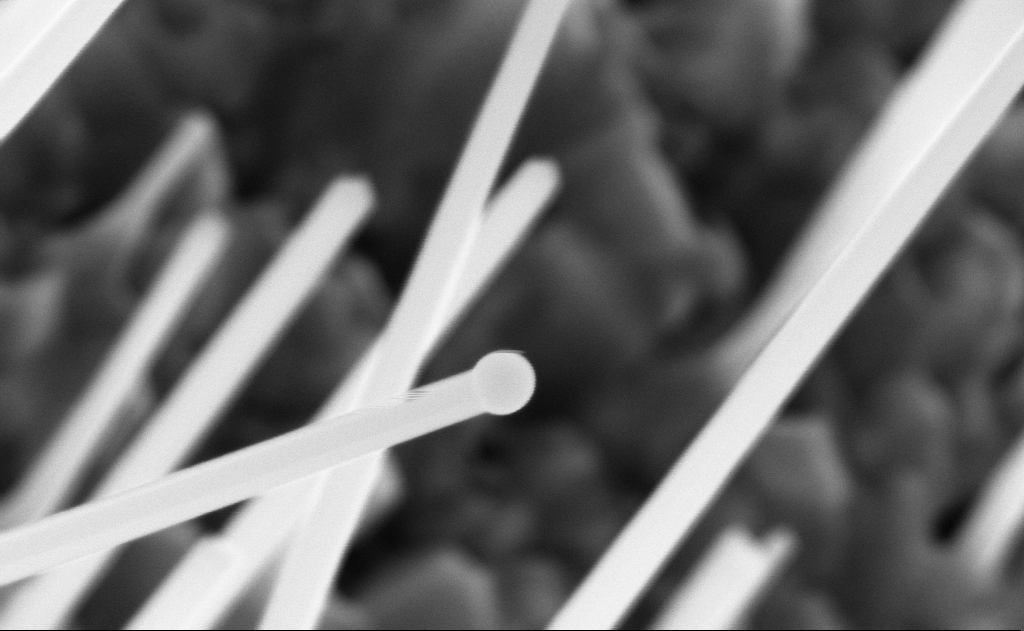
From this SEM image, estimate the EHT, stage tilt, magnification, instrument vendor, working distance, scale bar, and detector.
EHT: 10 kV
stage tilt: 0°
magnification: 150 K X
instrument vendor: Zeiss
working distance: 10 mm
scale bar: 200 nm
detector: InLens